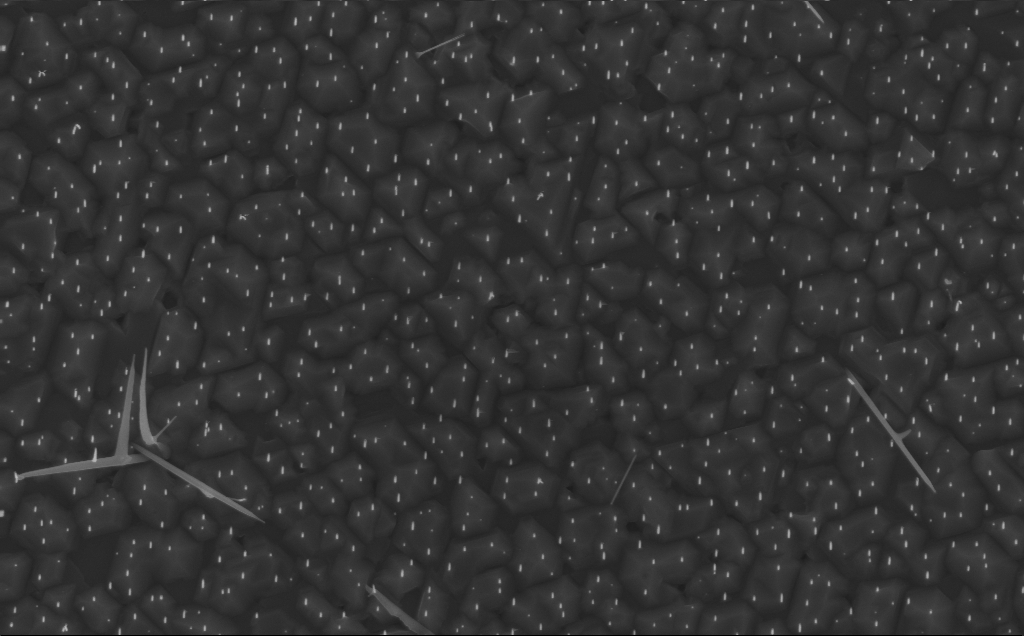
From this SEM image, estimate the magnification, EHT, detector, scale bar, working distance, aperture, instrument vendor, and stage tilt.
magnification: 20 K X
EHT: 10 kV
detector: InLens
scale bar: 2000 nm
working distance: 4 mm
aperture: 30 µm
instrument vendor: Zeiss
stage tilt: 0°